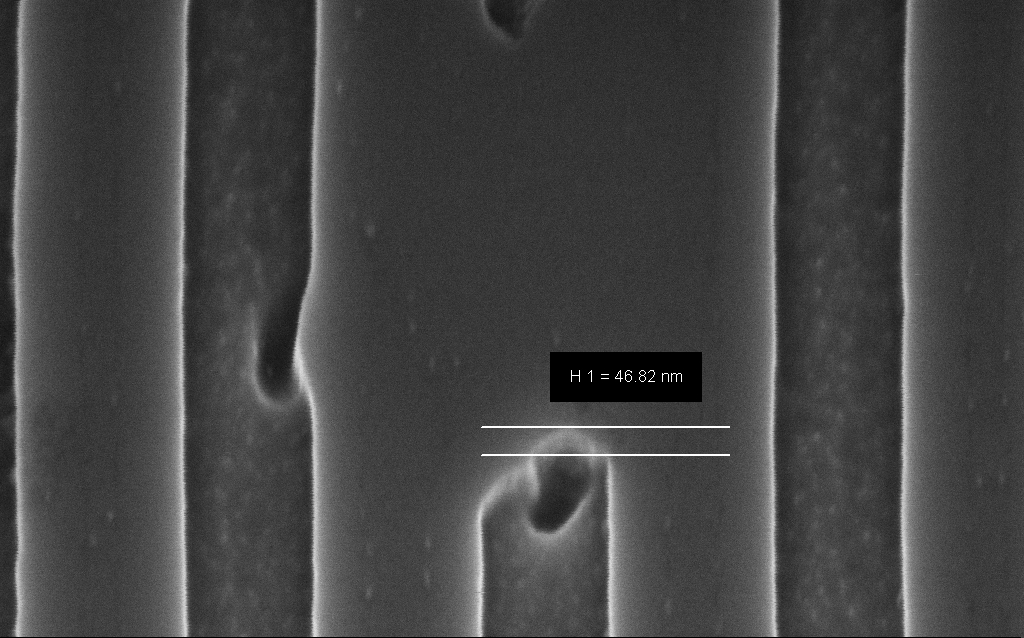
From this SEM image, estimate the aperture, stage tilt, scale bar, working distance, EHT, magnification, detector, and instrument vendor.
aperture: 30 µm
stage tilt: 45°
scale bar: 200 nm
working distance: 6 mm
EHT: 3 kV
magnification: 219.57 K X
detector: InLens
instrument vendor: Zeiss